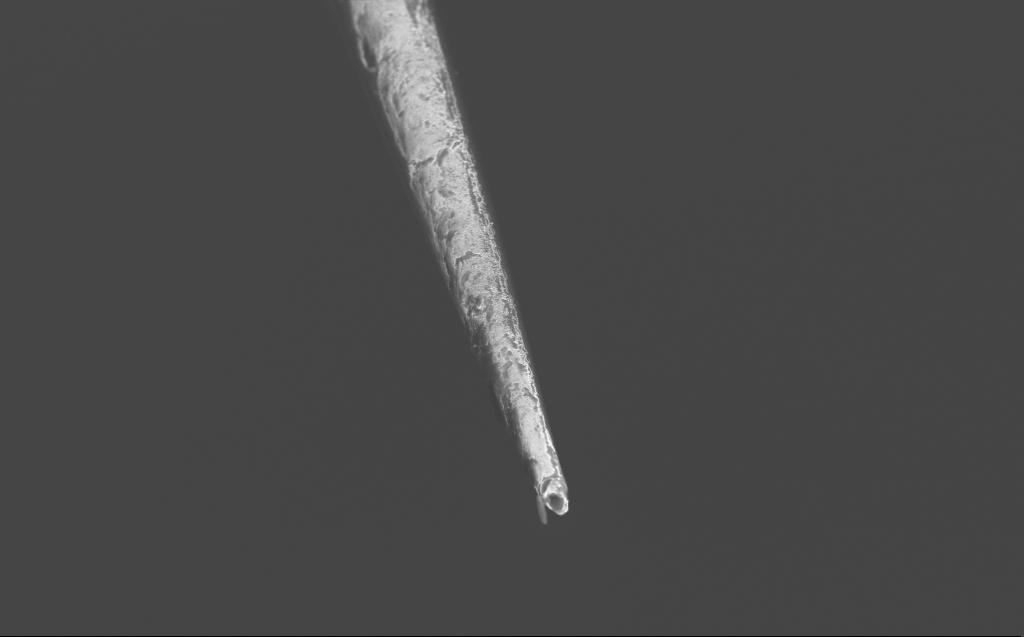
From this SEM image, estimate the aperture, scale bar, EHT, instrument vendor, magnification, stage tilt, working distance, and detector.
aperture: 30 µm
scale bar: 20000 nm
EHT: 3 kV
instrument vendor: Zeiss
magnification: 2.75 K X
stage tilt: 45°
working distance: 4 mm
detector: InLens